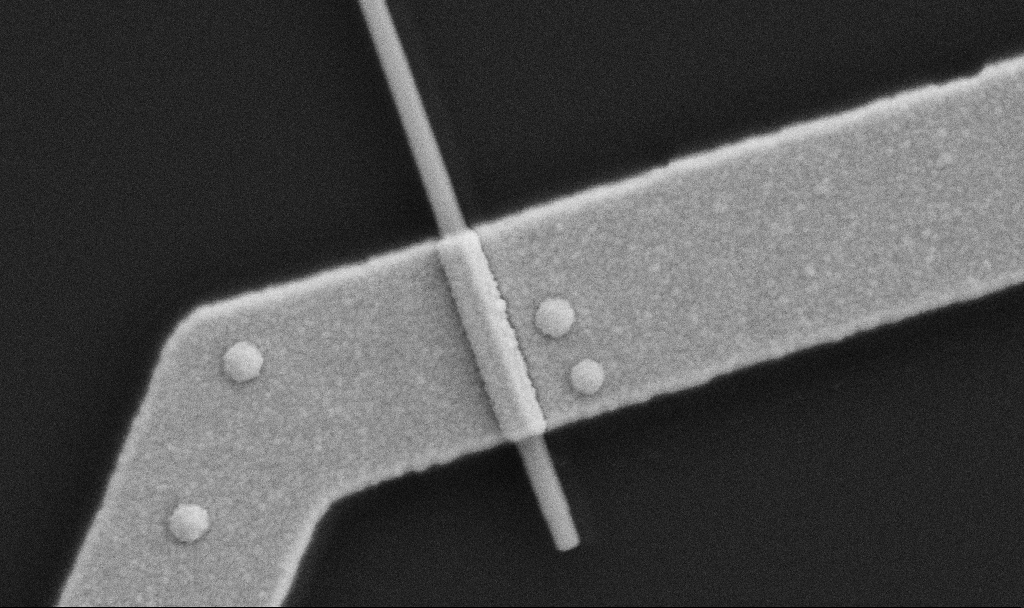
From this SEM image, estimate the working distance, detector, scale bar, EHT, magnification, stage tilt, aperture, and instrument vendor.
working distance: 10.7 mm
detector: SE2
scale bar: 200 nm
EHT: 5 kV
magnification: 100 K X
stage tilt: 0°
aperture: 30 µm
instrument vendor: Zeiss